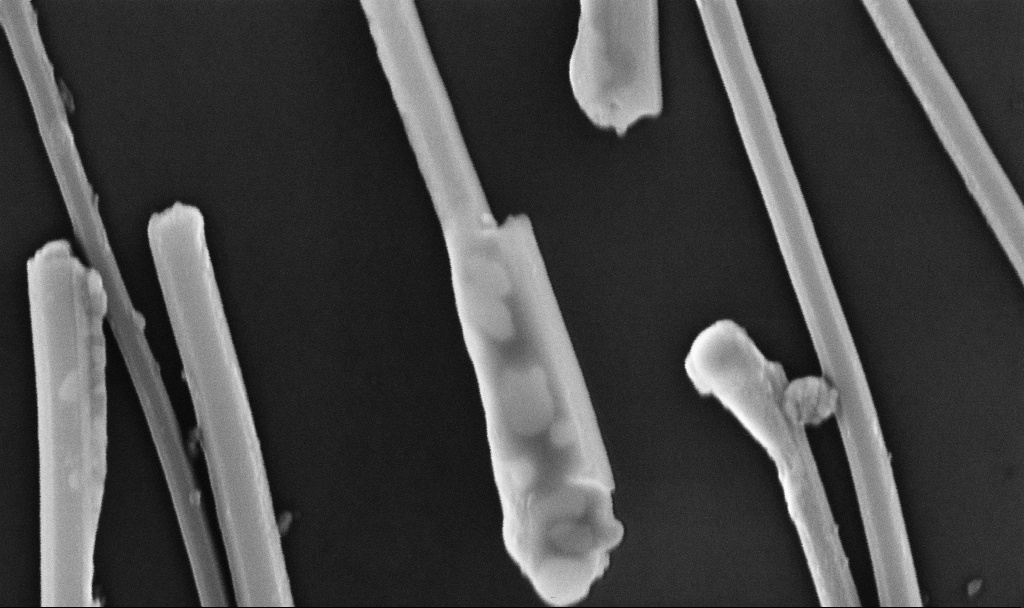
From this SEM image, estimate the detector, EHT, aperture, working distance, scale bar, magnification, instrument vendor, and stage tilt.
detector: InLens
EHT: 10 kV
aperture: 30 µm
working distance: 6.7 mm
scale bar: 200 nm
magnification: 143.39 K X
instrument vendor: Zeiss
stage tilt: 0°